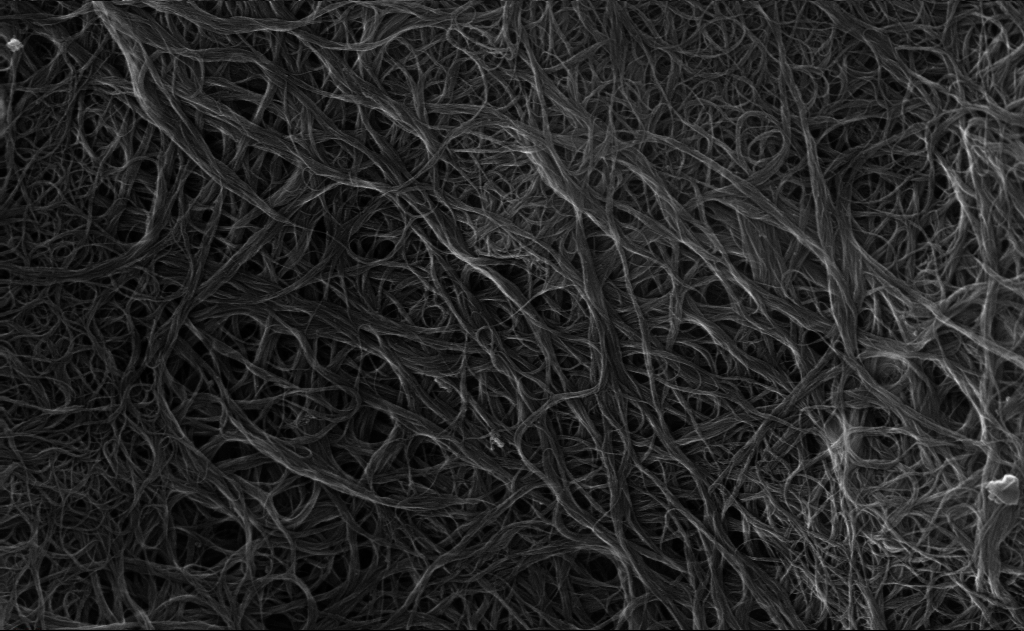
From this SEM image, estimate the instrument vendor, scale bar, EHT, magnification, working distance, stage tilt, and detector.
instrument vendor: Zeiss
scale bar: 200 nm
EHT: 10 kV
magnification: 85.03 K X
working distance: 3 mm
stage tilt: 0°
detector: InLens